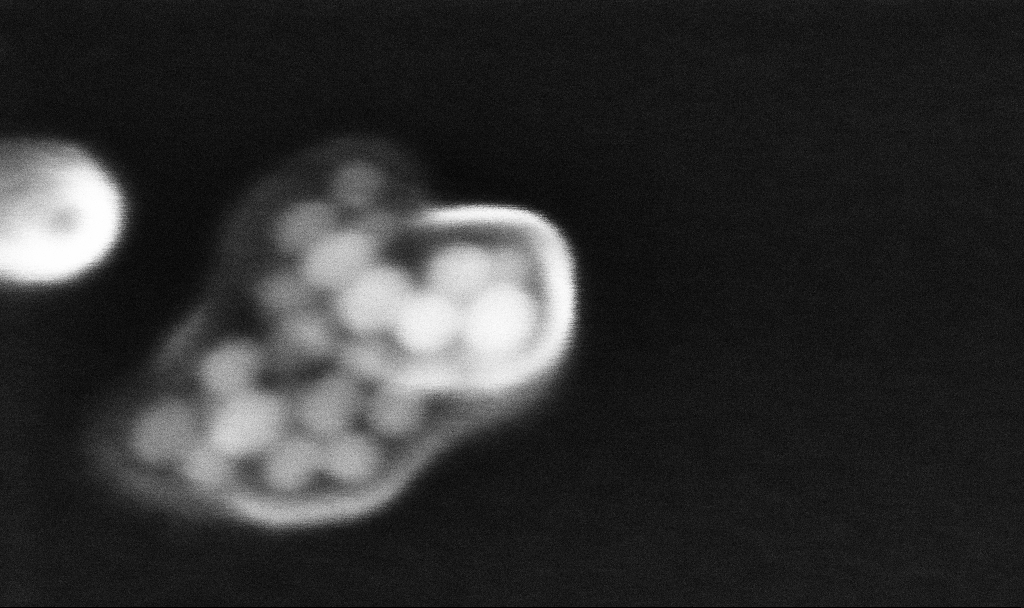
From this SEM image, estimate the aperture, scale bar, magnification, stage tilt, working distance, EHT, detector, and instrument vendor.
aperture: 30 µm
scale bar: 20 nm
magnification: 1192.45 K X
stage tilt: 0°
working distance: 3.3 mm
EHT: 10 kV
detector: InLens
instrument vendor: Zeiss